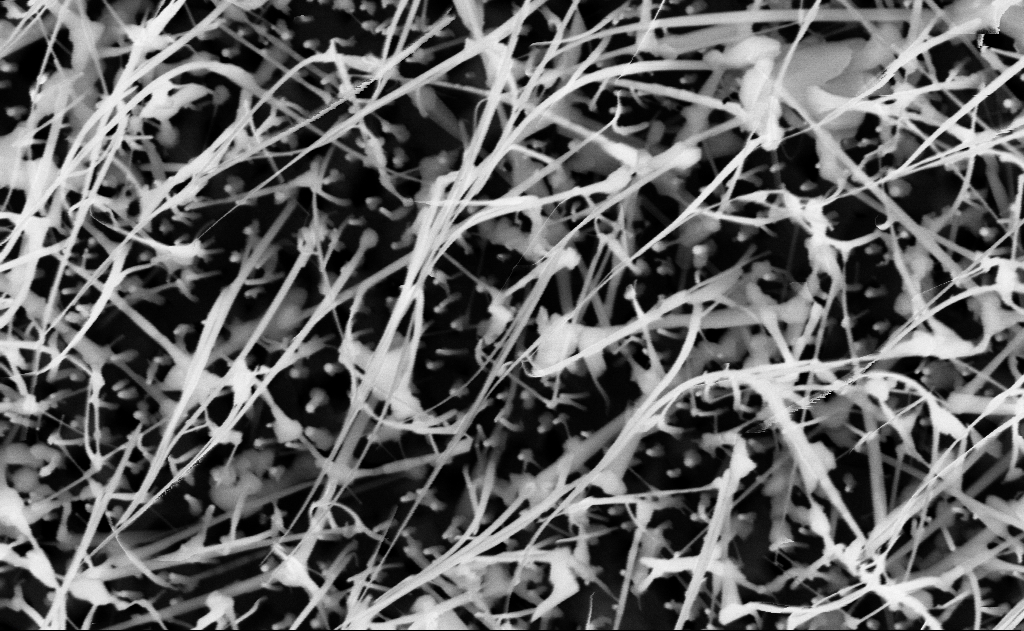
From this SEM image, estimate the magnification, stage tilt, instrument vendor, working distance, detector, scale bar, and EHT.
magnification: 60 K X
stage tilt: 0°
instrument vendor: Zeiss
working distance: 14 mm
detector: InLens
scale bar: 1000 nm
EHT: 10 kV